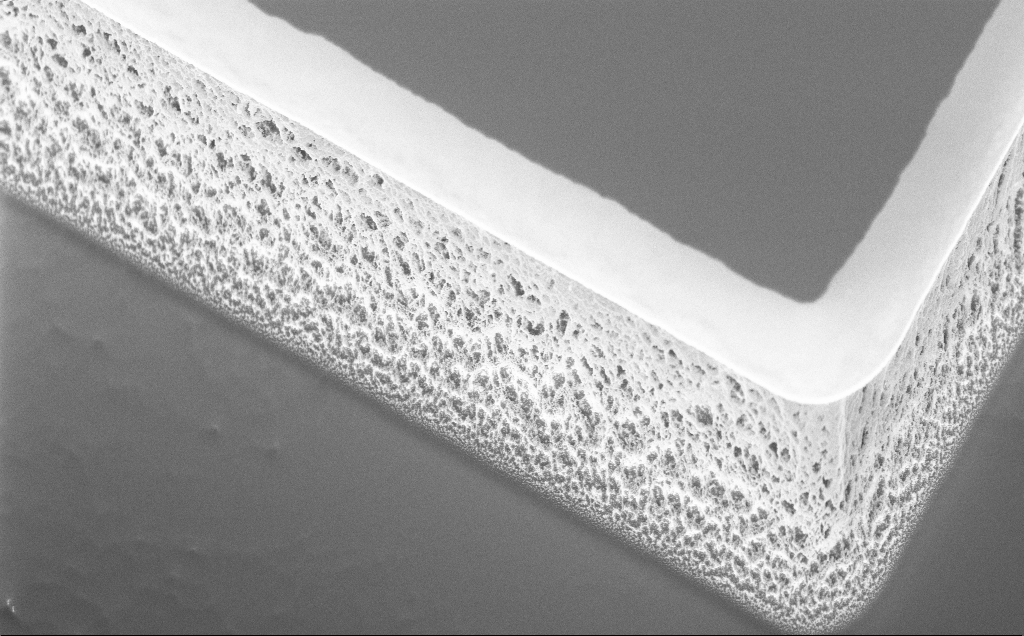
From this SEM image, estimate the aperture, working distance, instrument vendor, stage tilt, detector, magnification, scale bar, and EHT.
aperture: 30 µm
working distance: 8 mm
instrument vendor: Zeiss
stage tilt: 45°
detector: InLens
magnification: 7.69 K X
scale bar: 2000 nm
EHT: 5 kV